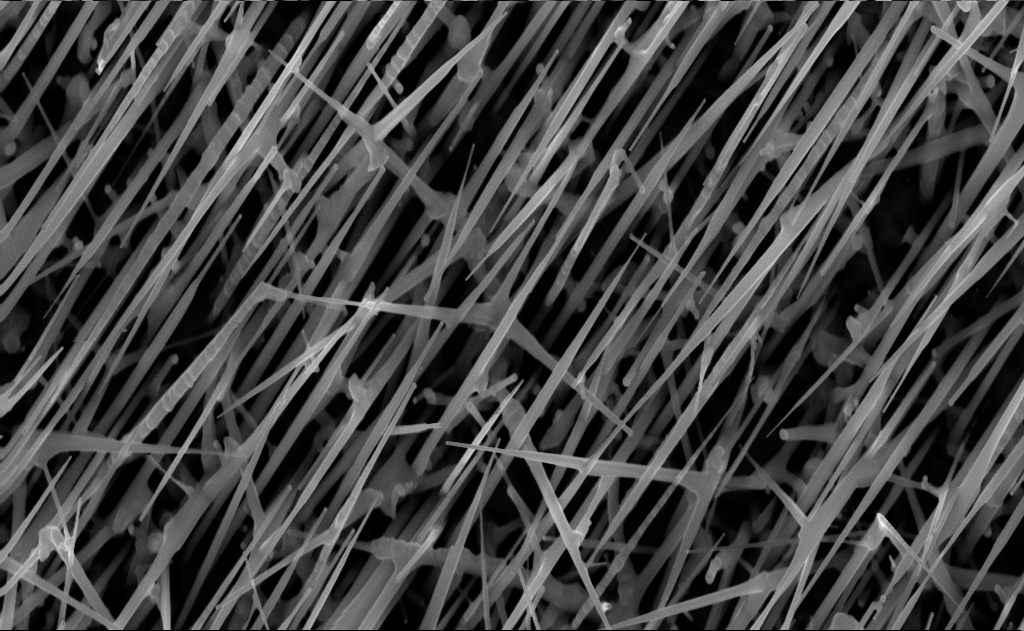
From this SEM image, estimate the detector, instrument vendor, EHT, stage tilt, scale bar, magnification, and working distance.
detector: InLens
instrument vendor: Zeiss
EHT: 10 kV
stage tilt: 0°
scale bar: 1000 nm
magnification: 40 K X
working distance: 7 mm